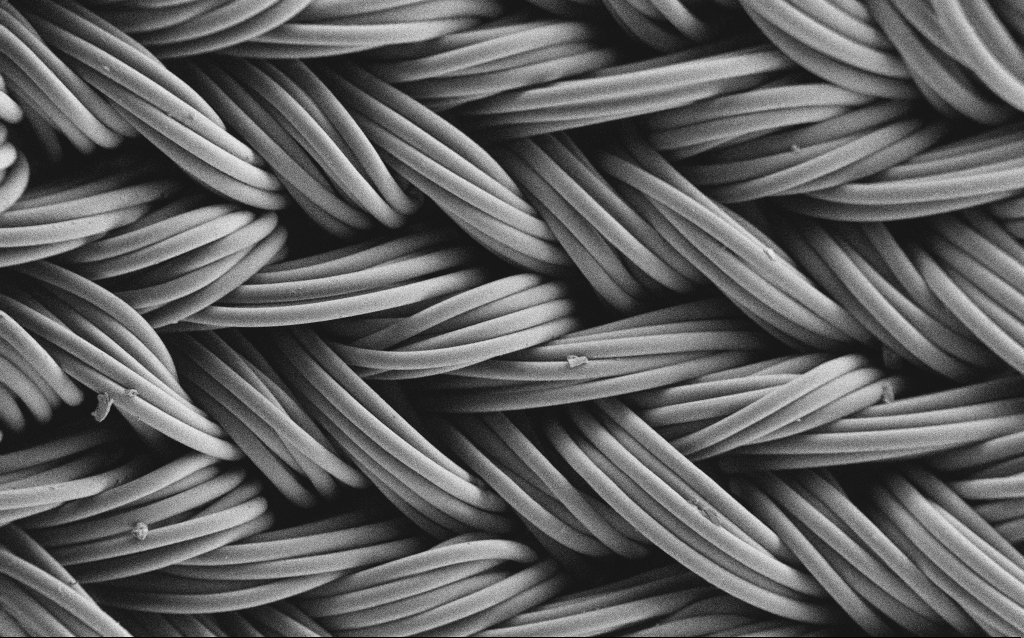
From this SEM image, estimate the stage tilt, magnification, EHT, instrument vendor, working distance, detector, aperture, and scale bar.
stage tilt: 0°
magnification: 0.29 K X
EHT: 1 kV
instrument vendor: Zeiss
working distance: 4 mm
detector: SE2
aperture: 30 µm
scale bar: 200000 nm